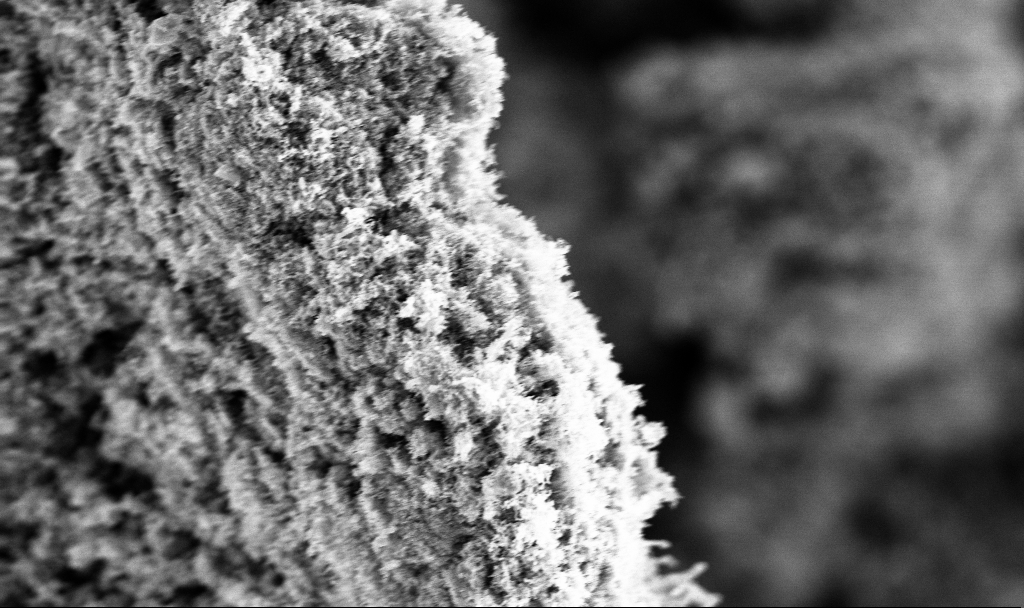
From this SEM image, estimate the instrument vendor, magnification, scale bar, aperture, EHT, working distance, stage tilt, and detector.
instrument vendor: Zeiss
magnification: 25 K X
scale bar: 1000 nm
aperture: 30 µm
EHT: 3 kV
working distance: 3.8 mm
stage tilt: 0°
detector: InLens